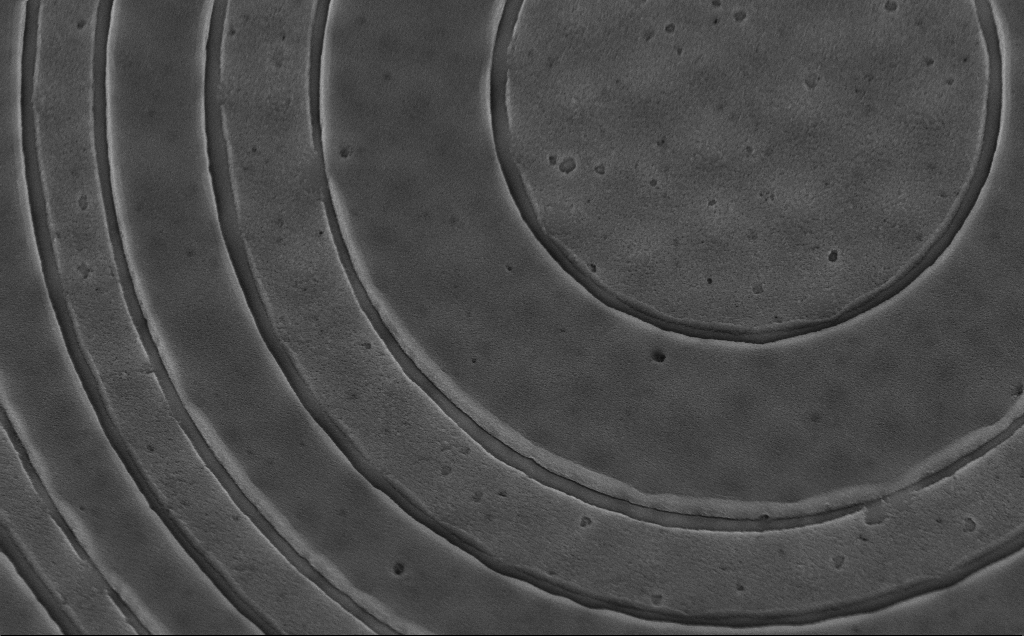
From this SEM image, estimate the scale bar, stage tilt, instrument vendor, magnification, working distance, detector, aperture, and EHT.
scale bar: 2000 nm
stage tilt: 45°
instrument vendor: Zeiss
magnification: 25.74 K X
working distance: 6 mm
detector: InLens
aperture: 30 µm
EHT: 5 kV